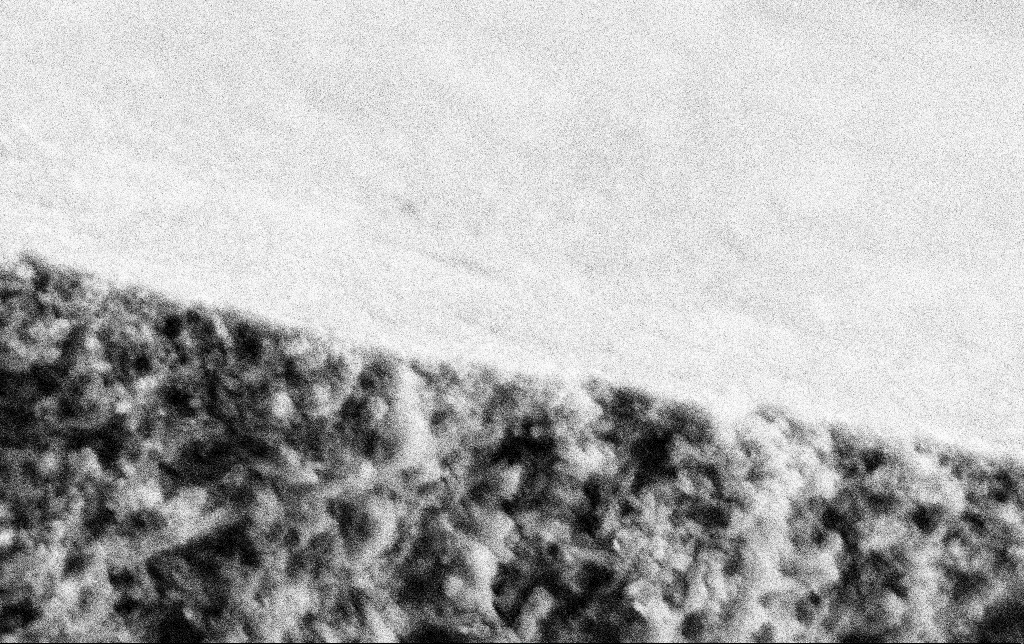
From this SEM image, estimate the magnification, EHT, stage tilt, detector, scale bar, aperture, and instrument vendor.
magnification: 100 K X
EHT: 2 kV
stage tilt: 0°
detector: SE2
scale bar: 200 nm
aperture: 30 µm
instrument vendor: Zeiss